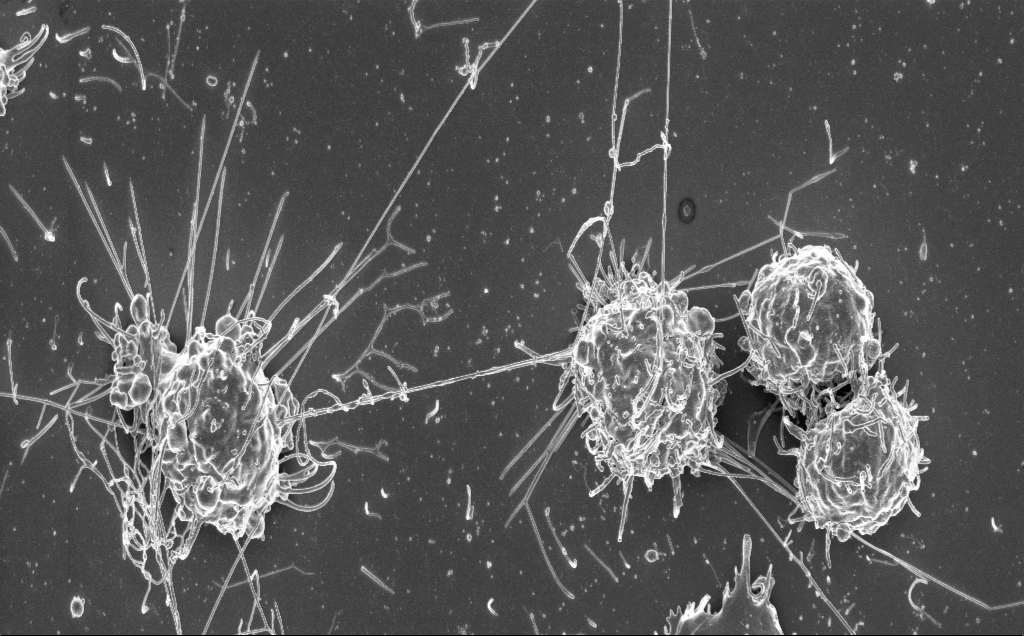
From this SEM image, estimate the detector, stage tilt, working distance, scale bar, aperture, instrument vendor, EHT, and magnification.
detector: InLens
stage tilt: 0.8°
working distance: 6 mm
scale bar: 10000 nm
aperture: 30 µm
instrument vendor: Zeiss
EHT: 3 kV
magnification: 6.16 K X